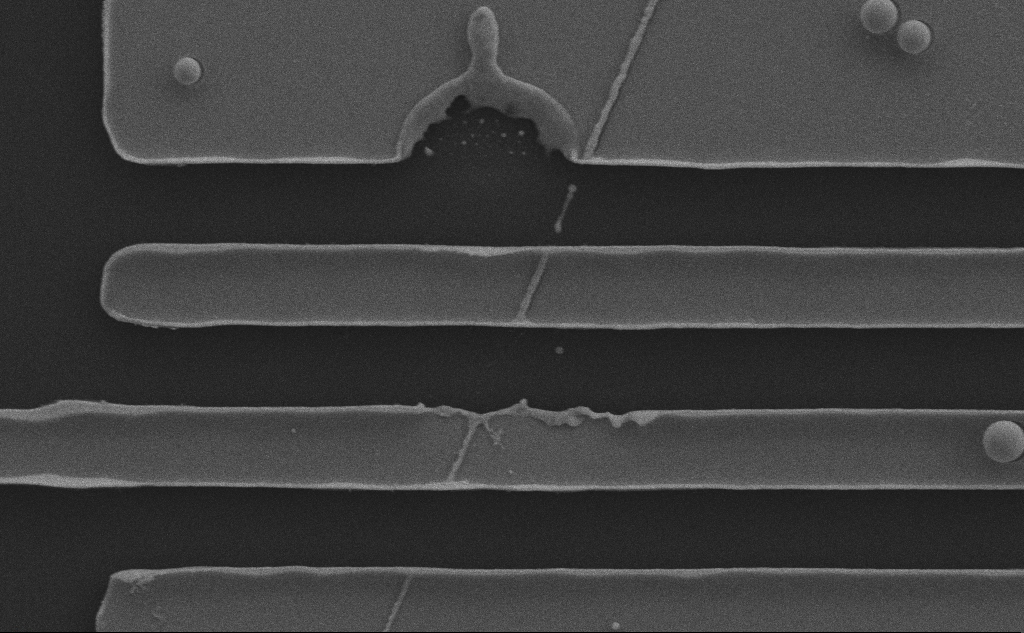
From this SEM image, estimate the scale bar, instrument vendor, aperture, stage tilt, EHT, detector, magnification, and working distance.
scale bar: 2000 nm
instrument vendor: Zeiss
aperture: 30 µm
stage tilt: -0.1°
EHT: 10 kV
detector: SE2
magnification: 14.97 K X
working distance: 6 mm